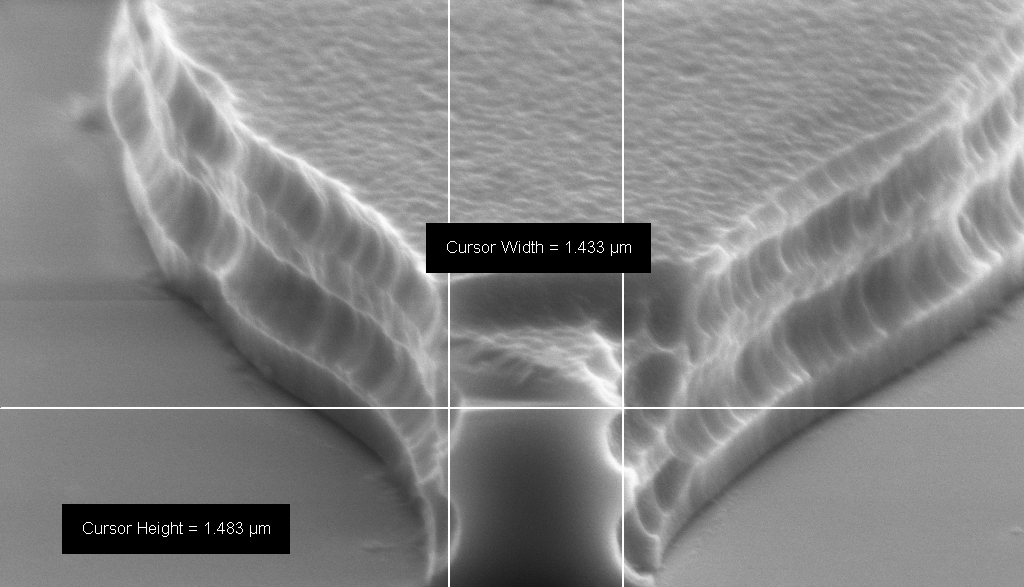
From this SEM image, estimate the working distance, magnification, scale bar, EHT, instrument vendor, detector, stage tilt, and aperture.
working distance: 12 mm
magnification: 44.58 K X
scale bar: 1000 nm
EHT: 8 kV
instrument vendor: Zeiss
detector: SE2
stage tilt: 70°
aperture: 30 µm